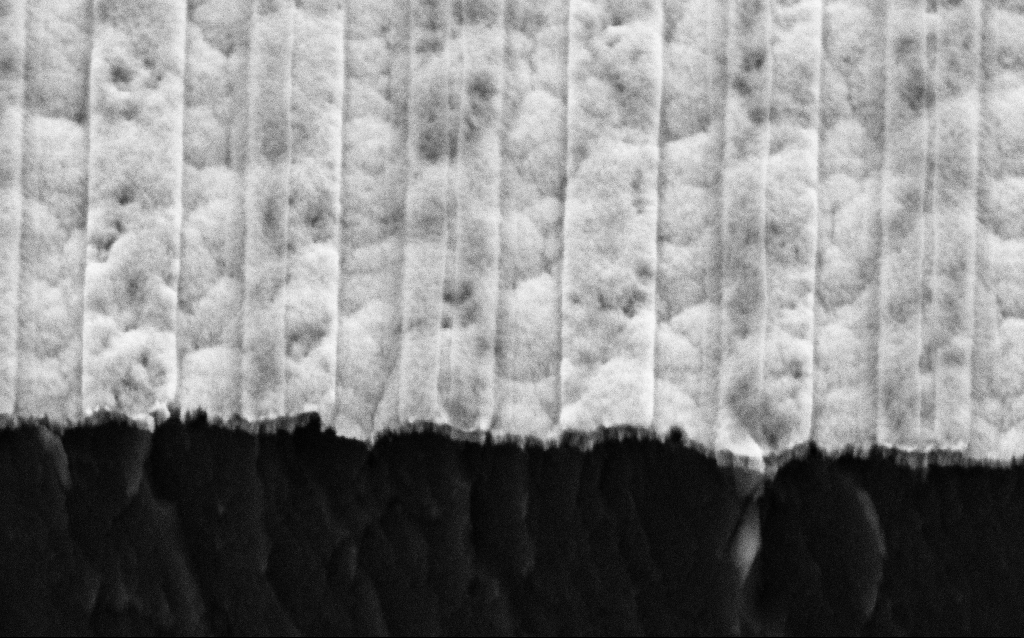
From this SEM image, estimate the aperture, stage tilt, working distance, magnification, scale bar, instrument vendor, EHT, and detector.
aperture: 30 µm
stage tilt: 45°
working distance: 6 mm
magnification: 118.07 K X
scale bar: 200 nm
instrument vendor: Zeiss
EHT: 3 kV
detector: SE2